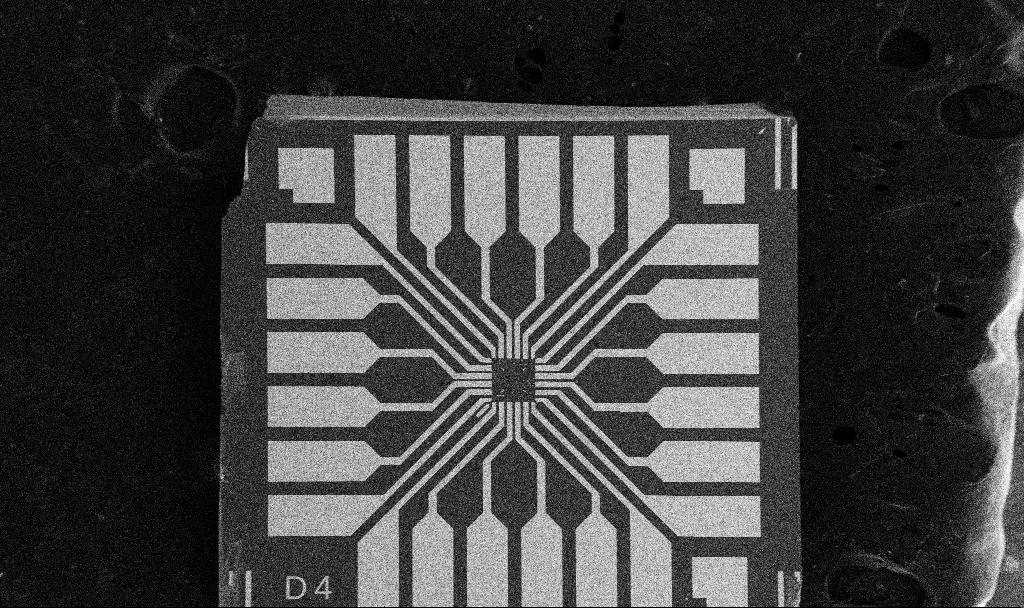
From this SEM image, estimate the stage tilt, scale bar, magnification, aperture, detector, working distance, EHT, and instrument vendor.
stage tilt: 0°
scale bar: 200000 nm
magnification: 0.1 K X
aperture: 30 µm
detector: SE2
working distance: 10.6 mm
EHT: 5 kV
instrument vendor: Zeiss